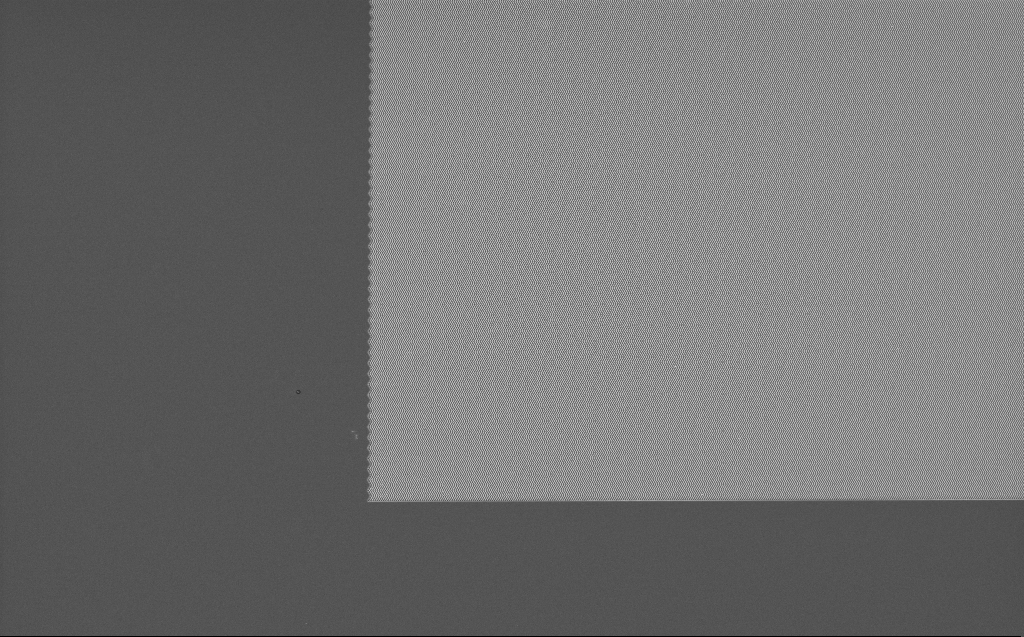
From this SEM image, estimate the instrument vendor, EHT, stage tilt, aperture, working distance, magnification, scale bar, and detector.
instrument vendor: Zeiss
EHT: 5 kV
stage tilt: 0°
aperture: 30 µm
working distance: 7 mm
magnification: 1.97 K X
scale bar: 20000 nm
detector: InLens